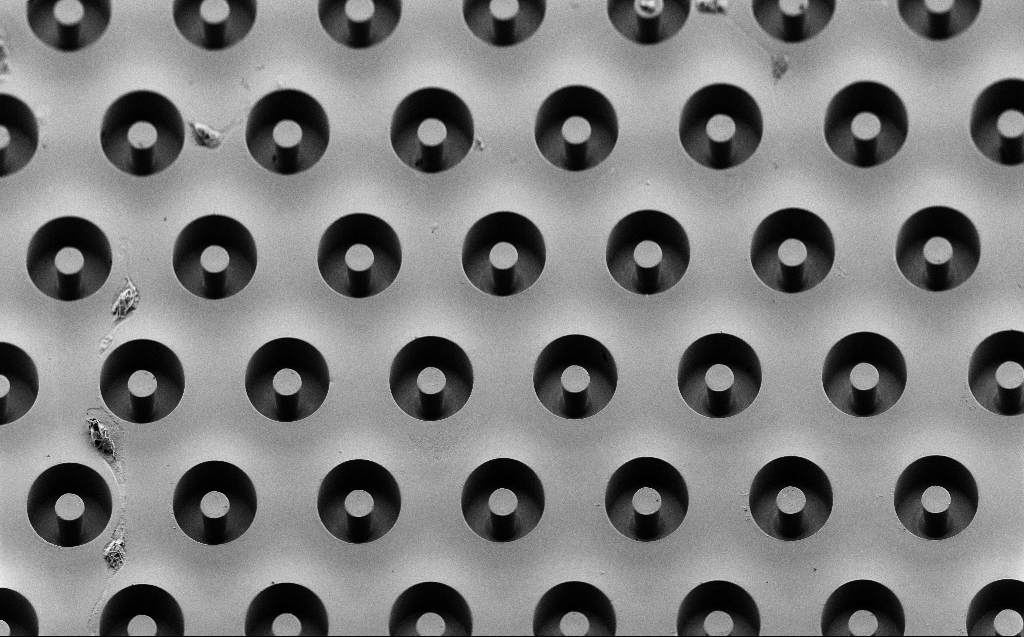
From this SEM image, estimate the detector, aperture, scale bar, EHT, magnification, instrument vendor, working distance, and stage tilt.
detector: SE2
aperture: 30 µm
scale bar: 100000 nm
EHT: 2 kV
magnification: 0.496 K X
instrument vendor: Zeiss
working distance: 6 mm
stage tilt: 45°